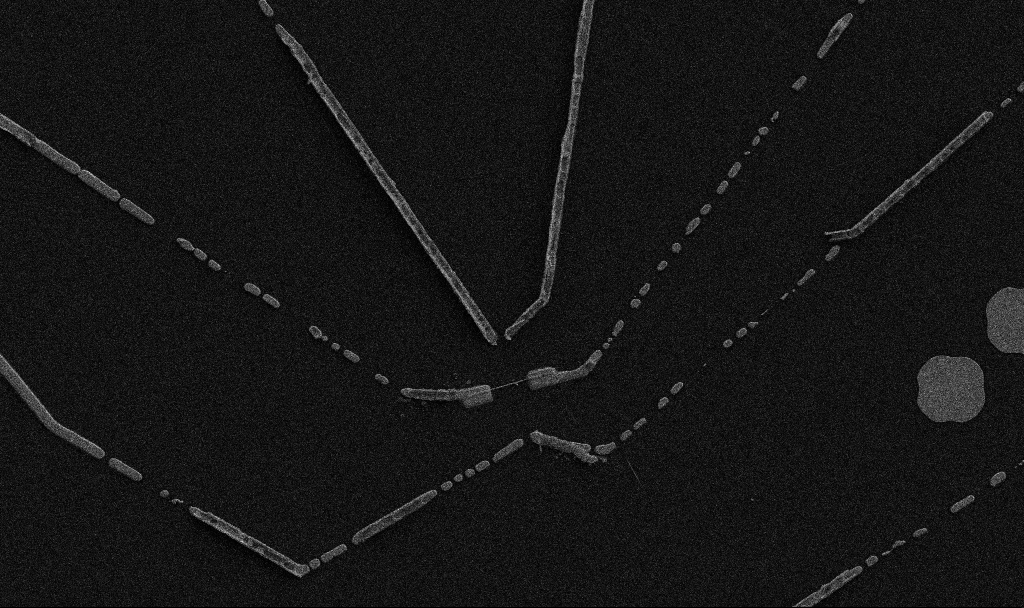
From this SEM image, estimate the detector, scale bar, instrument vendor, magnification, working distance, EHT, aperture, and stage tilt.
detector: SE2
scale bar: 10000 nm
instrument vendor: Zeiss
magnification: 5 K X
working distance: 10.7 mm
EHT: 5 kV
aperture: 30 µm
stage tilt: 0°